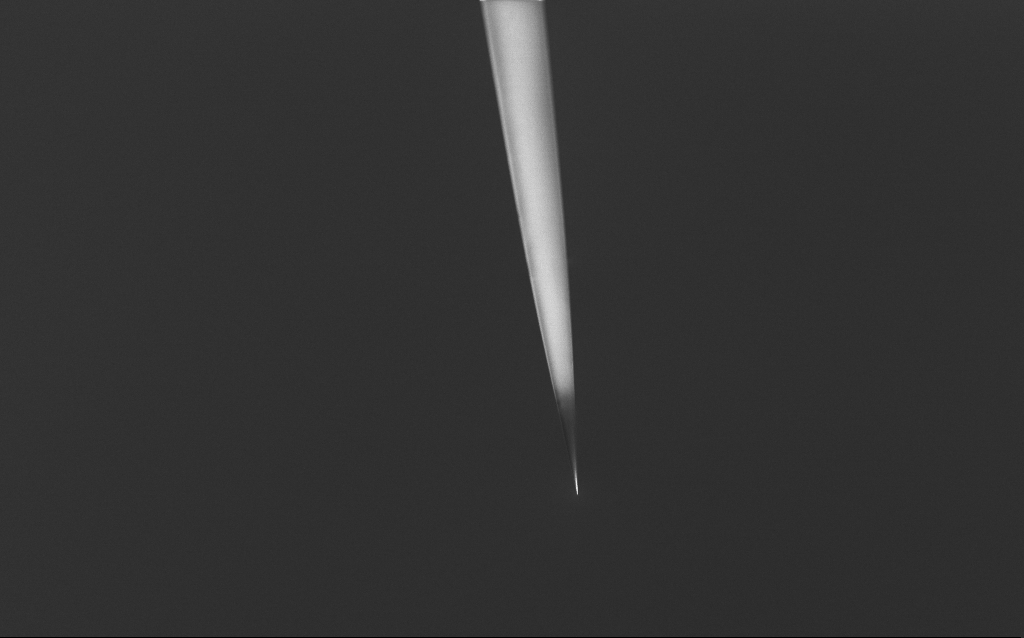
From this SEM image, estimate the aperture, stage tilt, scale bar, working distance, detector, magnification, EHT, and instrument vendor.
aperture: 30 µm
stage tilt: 45°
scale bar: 20000 nm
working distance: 5 mm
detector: InLens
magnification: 1 K X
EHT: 2.5 kV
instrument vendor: Zeiss